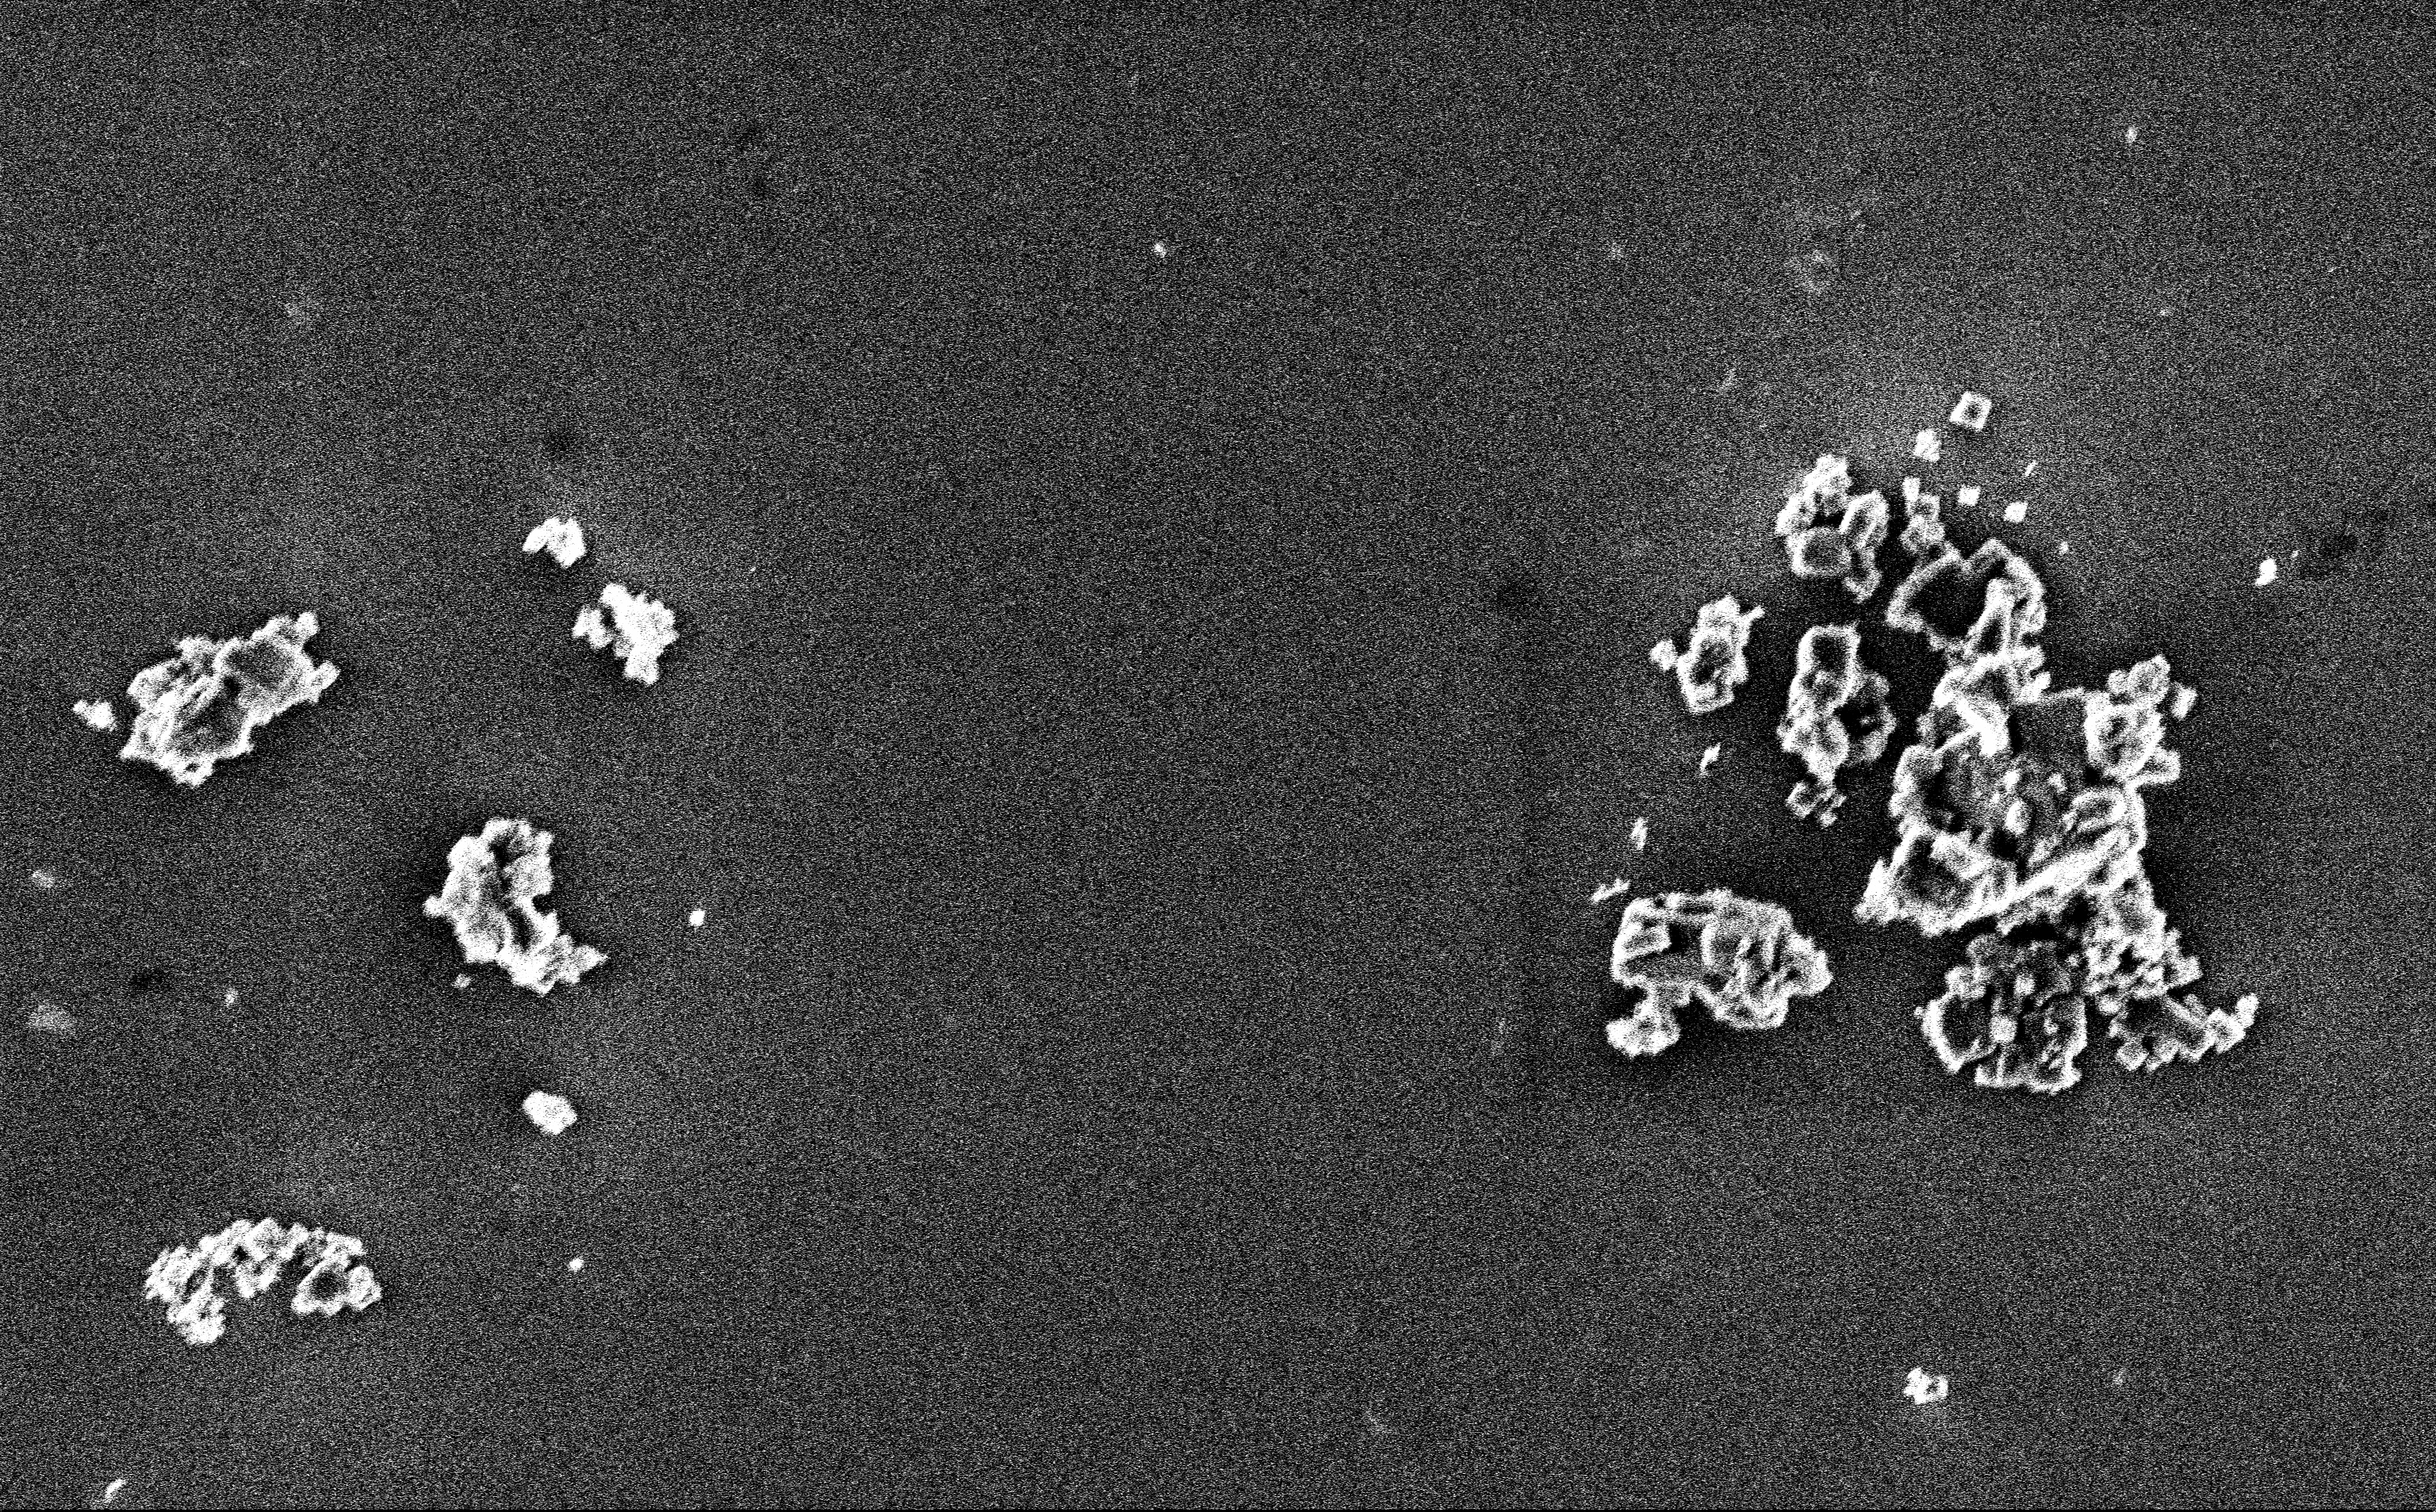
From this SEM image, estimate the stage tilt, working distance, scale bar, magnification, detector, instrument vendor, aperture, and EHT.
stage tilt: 0°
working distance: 3 mm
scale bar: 2000 nm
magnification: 19.01 K X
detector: InLens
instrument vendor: Zeiss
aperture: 30 µm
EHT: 3 kV